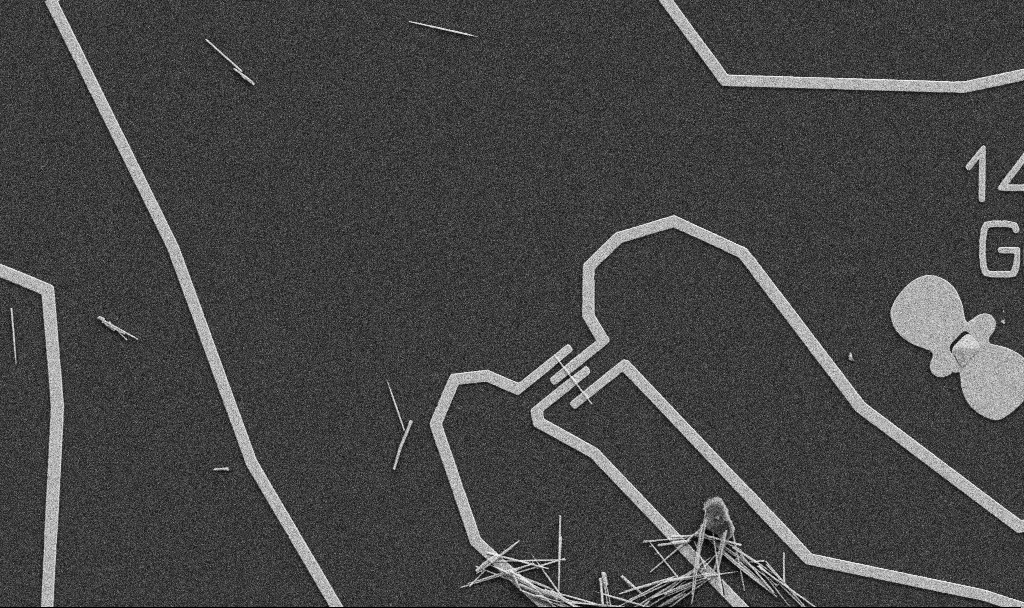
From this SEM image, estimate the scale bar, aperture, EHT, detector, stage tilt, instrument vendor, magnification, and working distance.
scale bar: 10000 nm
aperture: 30 µm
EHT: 5 kV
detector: SE2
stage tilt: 0°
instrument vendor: Zeiss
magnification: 5 K X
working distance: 10.7 mm